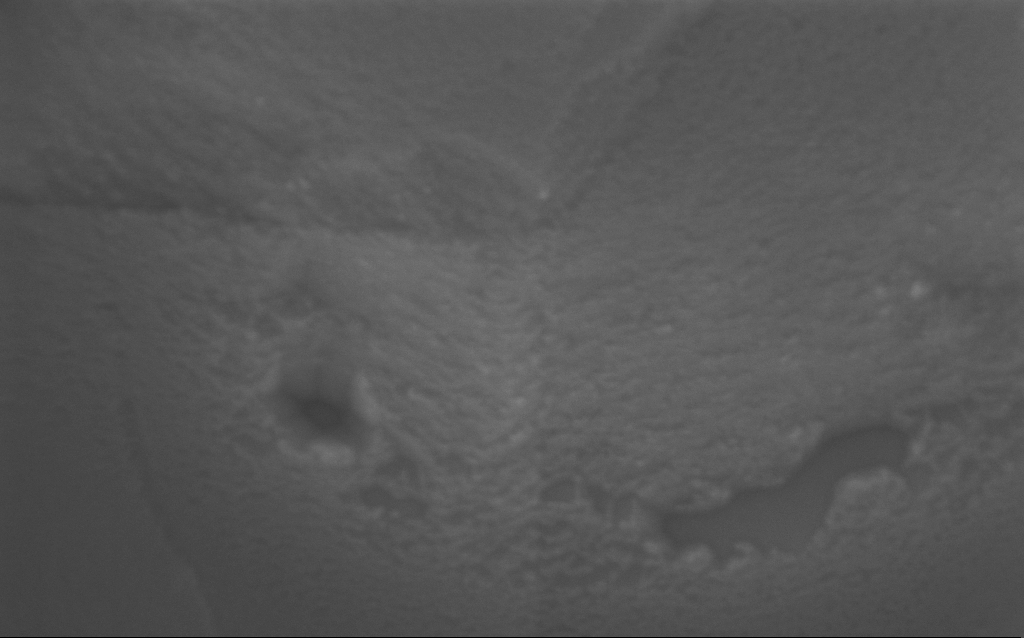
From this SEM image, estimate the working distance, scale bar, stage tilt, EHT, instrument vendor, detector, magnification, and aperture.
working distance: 2 mm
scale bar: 100 nm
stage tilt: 0°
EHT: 10 kV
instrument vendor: Zeiss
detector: InLens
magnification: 489.16 K X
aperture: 30 µm